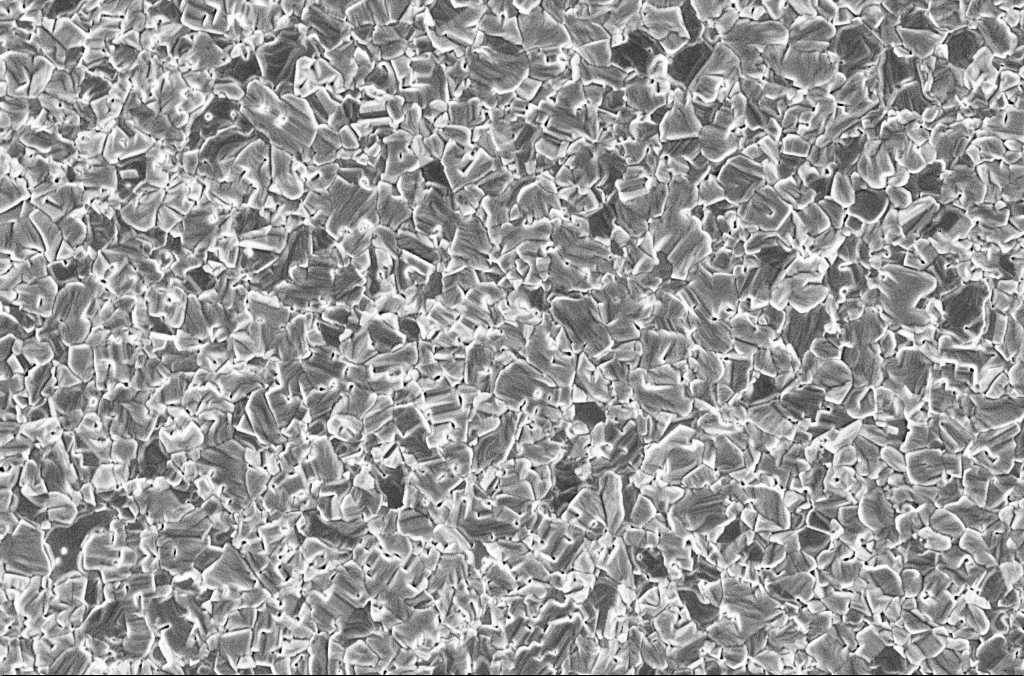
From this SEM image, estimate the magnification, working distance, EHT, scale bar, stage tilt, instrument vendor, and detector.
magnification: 20 K X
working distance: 3 mm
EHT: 2 kV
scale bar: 2000 nm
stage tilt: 0°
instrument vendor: Zeiss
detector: InLens